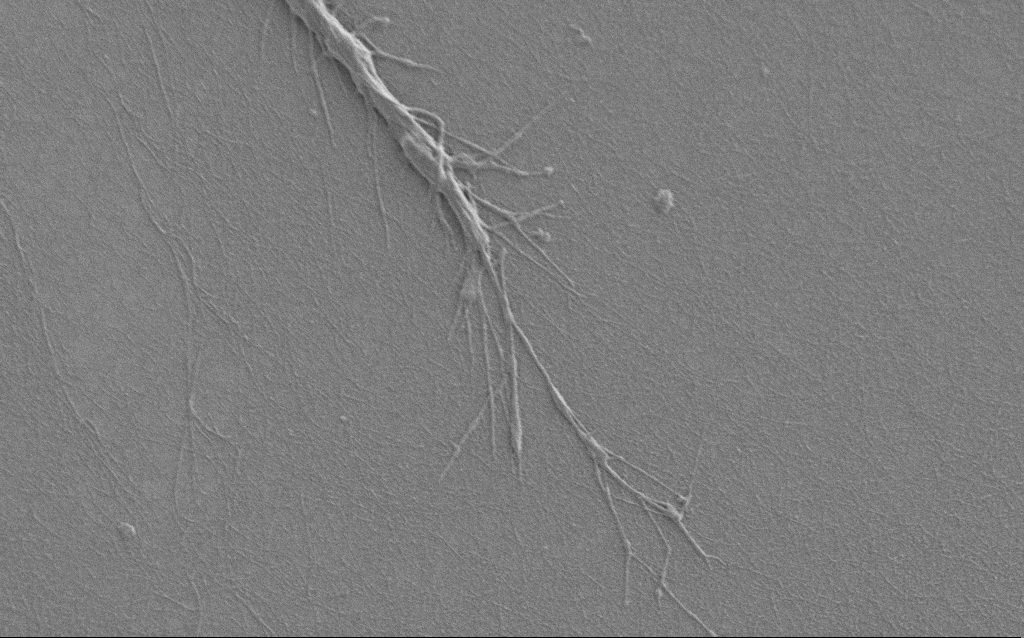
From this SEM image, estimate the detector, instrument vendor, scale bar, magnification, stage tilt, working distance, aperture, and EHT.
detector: SE2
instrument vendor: Zeiss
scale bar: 10000 nm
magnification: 6.5 K X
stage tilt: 0°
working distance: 6 mm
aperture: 30 µm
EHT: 1 kV